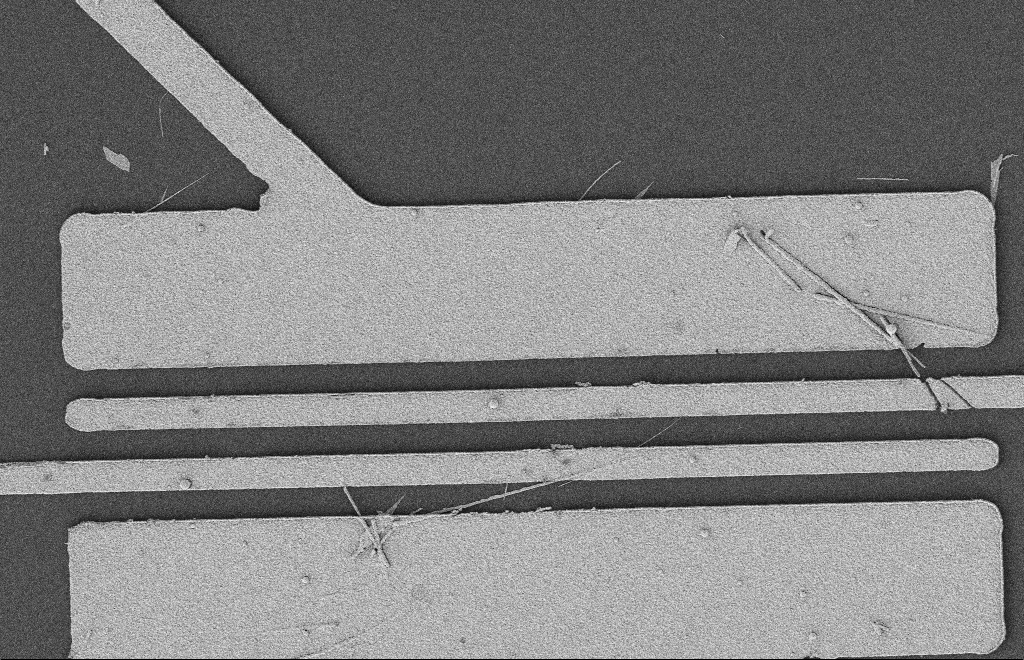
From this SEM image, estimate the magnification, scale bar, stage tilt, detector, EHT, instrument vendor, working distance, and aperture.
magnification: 5.59 K X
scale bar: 2000 nm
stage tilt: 0°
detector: SE2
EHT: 2 kV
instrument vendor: Zeiss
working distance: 12 mm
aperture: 20 µm